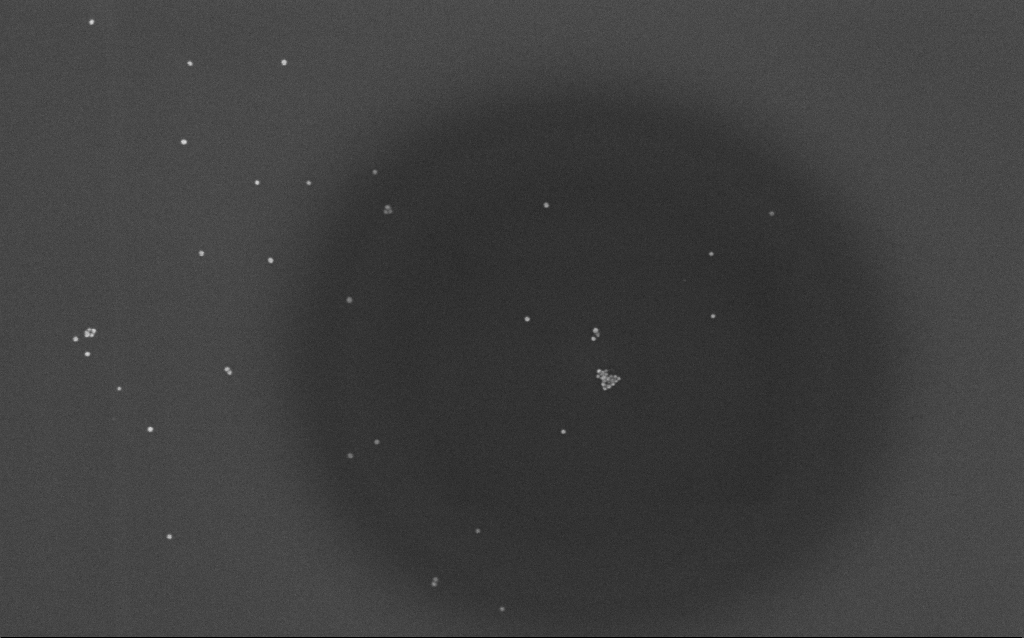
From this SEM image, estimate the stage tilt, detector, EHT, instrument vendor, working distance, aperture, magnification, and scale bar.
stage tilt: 0°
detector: InLens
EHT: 10 kV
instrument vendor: Zeiss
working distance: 7 mm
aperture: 30 µm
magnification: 100 K X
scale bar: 200 nm